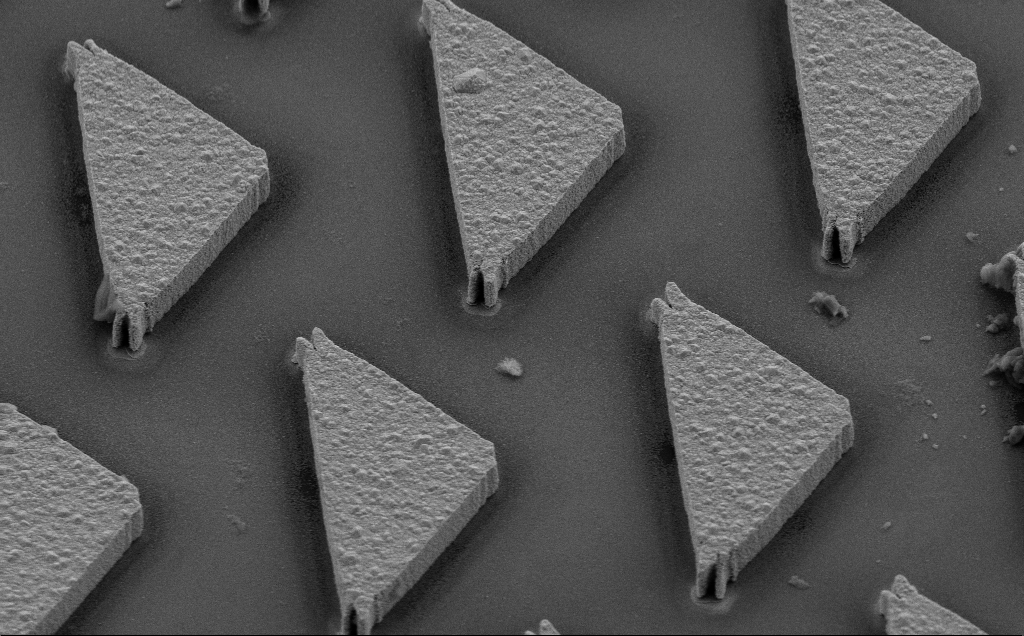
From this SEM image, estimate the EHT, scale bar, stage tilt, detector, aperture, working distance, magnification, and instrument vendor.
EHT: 10 kV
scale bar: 10000 nm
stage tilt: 35°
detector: SE2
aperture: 30 µm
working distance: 8 mm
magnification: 3.67 K X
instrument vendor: Zeiss